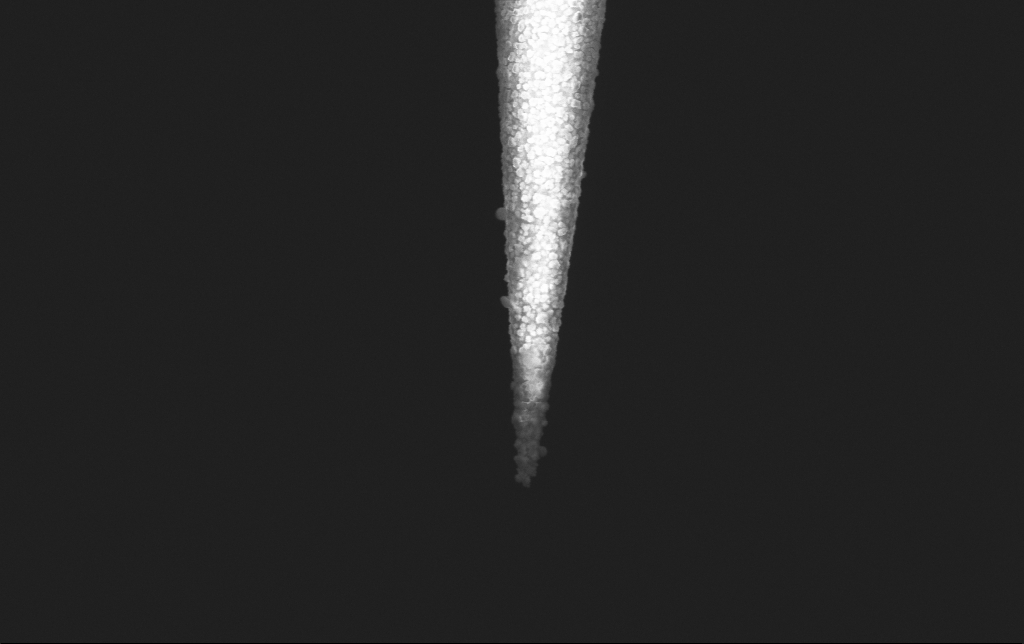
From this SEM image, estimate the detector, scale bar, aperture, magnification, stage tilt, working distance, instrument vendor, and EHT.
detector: InLens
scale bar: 1000 nm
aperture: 30 µm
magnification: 50 K X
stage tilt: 0°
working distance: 5.9 mm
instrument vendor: Zeiss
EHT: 2 kV